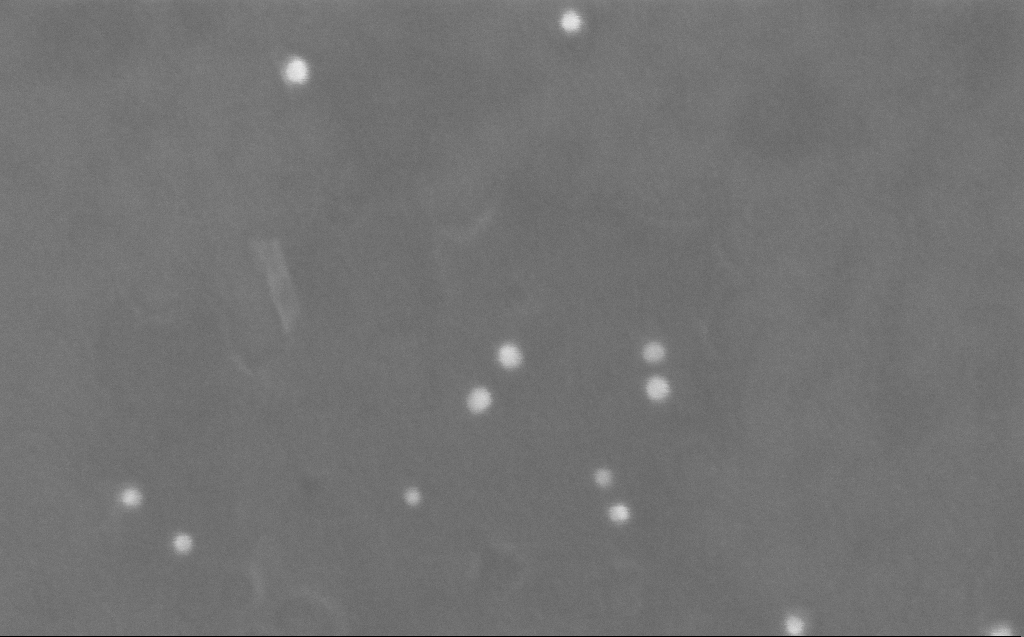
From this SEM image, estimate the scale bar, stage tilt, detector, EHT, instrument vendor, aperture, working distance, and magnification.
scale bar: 100 nm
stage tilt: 0°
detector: InLens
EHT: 10 kV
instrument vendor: Zeiss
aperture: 30 µm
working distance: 3 mm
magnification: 445.62 K X